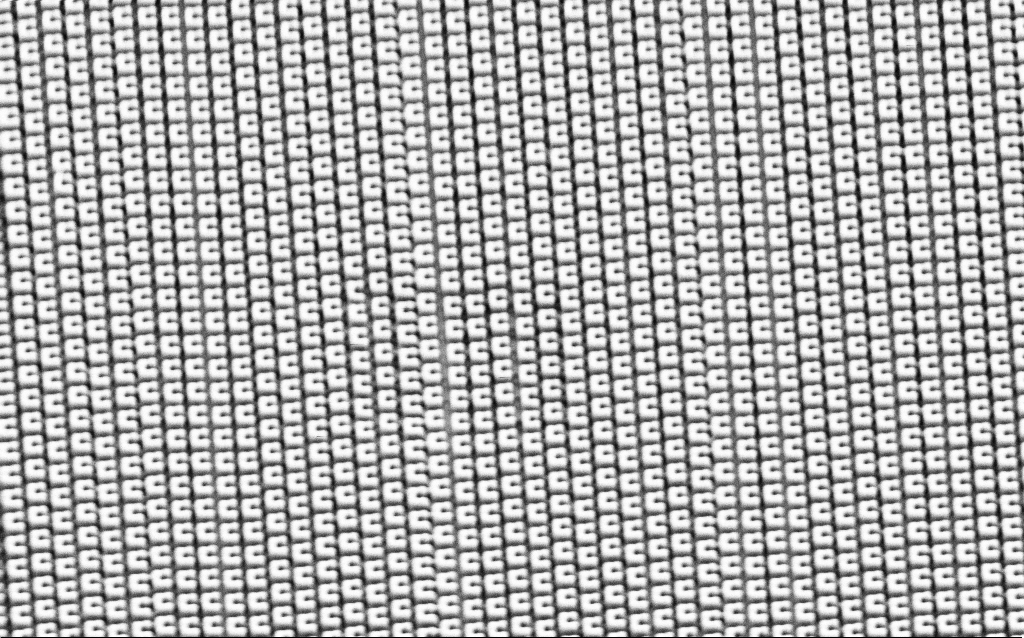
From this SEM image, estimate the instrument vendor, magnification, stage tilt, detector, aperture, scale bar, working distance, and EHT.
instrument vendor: Zeiss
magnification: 18.68 K X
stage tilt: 30°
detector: SE2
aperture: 30 µm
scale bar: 2000 nm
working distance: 6.4 mm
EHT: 1.5 kV